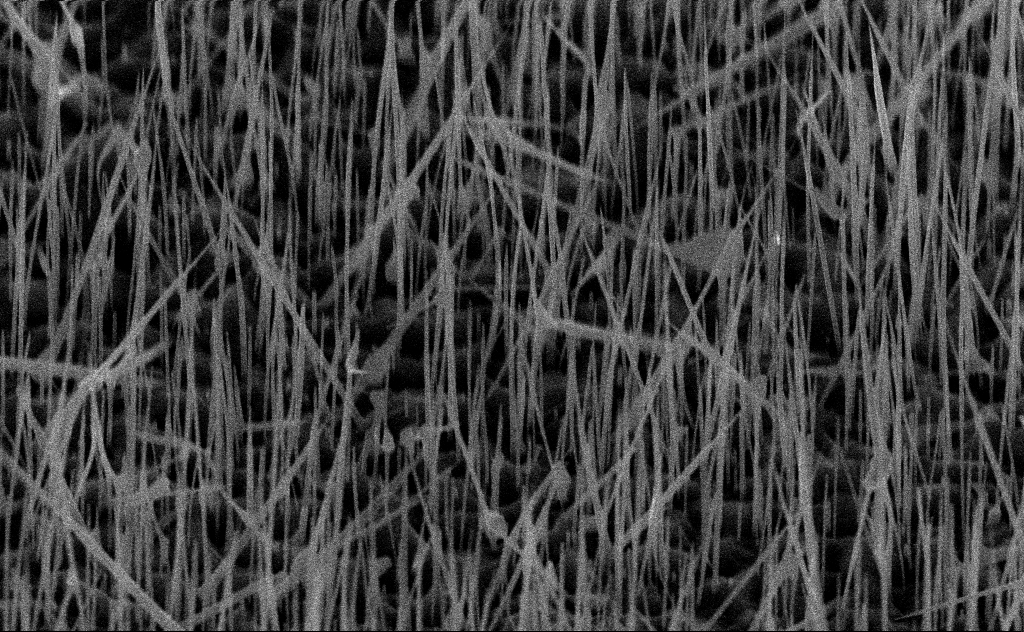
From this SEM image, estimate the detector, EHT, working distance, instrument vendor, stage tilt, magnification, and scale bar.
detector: InLens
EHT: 10 kV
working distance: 6 mm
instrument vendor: Zeiss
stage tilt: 44.2°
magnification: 40 K X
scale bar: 1000 nm